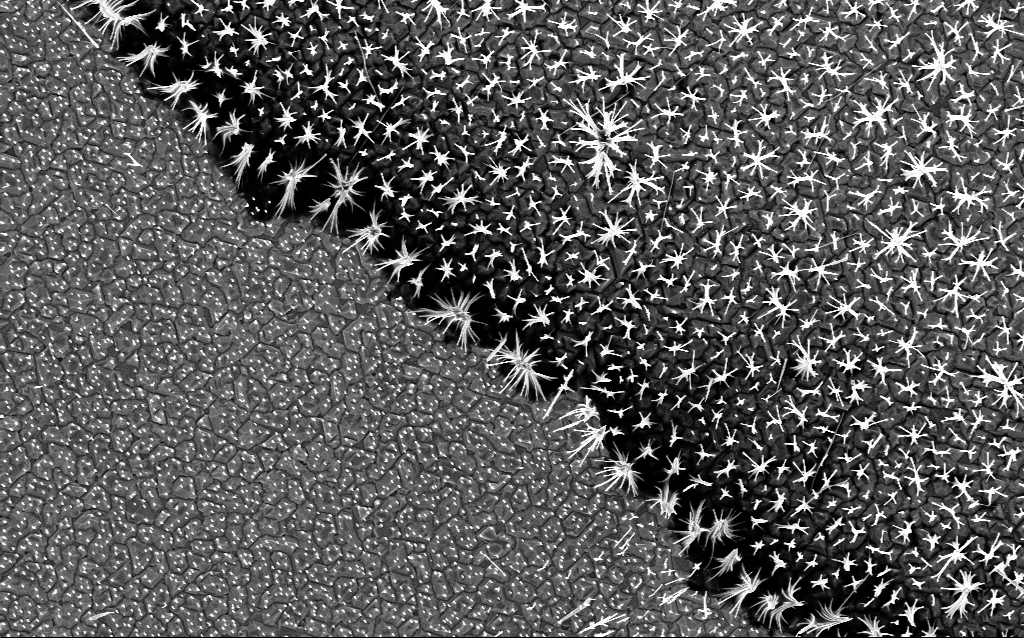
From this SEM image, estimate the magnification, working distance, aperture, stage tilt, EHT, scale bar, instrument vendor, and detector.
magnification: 6.78 K X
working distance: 6.6 mm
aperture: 30 µm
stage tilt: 0°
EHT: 5 kV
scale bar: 10000 nm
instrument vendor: Zeiss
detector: InLens